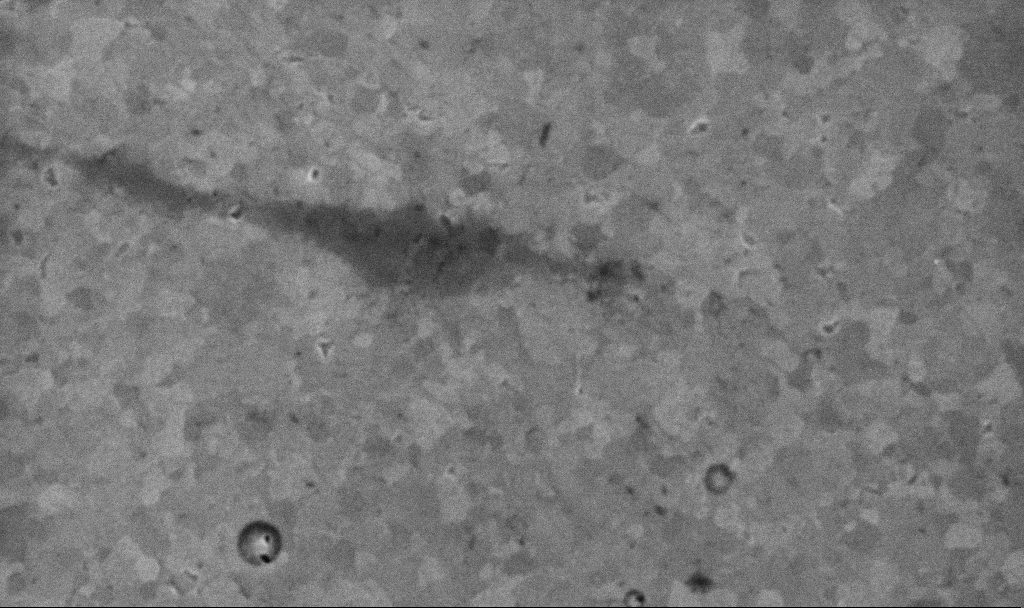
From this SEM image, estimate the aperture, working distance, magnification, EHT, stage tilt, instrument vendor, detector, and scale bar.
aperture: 30 µm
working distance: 3.1 mm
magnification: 50.56 K X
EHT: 10 kV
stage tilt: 0°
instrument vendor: Zeiss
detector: InLens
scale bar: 1000 nm